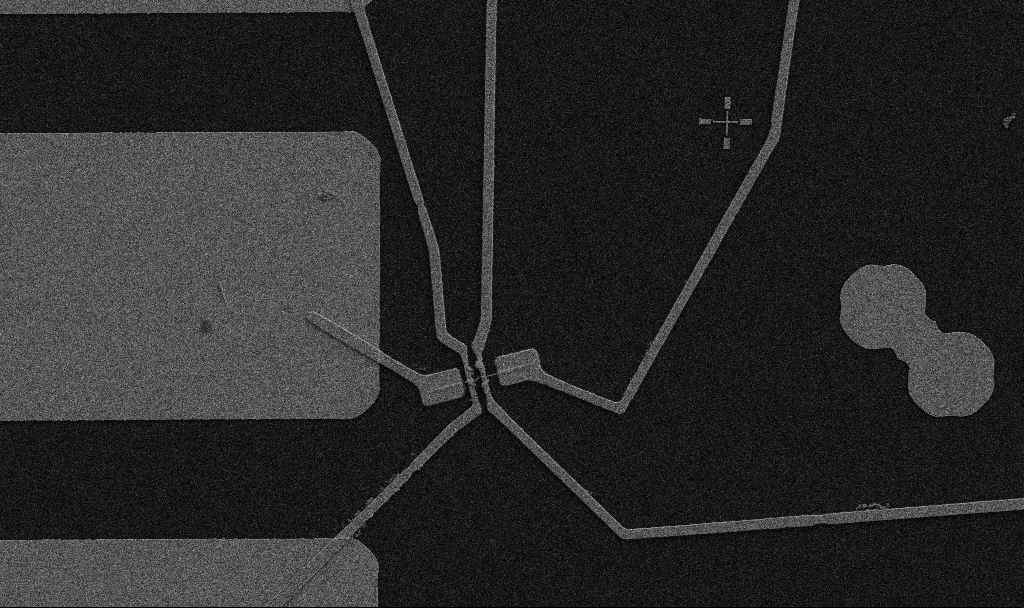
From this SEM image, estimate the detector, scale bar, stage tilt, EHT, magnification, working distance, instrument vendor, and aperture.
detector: SE2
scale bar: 10000 nm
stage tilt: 0°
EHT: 5 kV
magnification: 5 K X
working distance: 10.7 mm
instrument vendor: Zeiss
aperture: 30 µm